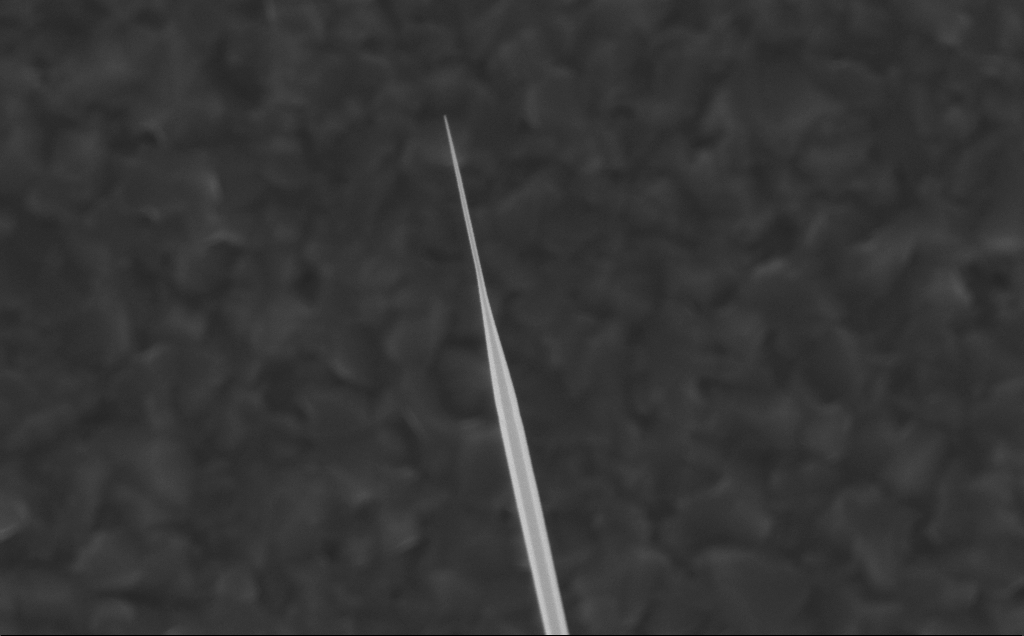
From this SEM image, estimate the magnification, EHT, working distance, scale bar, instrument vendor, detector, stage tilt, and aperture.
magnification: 61.26 K X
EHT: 10 kV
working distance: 5 mm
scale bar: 1000 nm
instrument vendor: Zeiss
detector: InLens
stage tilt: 0°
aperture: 30 µm